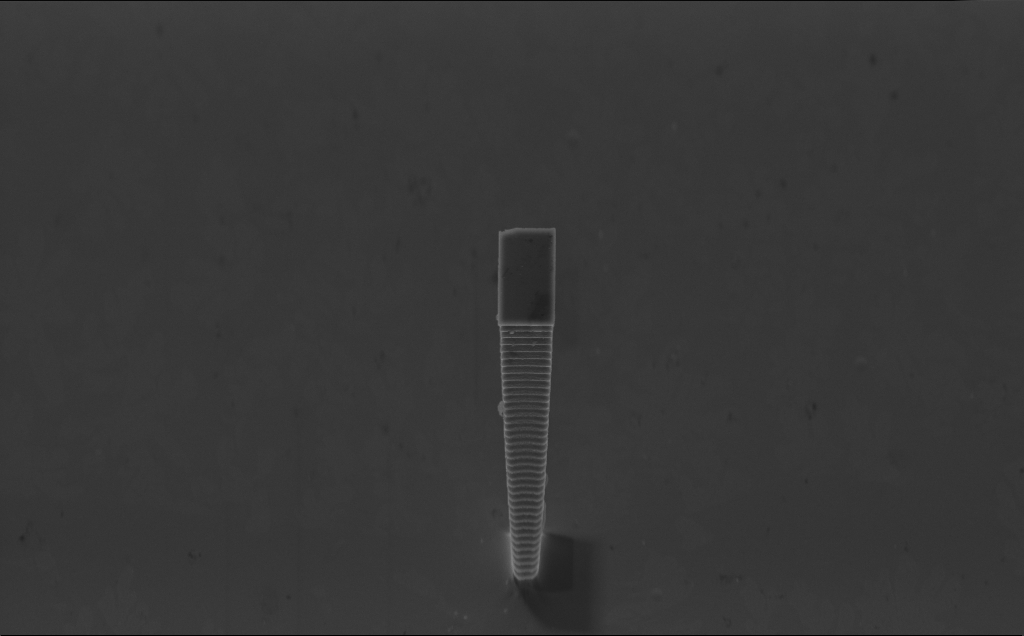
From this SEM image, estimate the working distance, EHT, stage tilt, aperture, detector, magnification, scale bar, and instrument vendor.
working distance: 7 mm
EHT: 5 kV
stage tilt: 45°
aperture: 30 µm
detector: InLens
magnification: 7.23 K X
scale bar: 10000 nm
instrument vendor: Zeiss